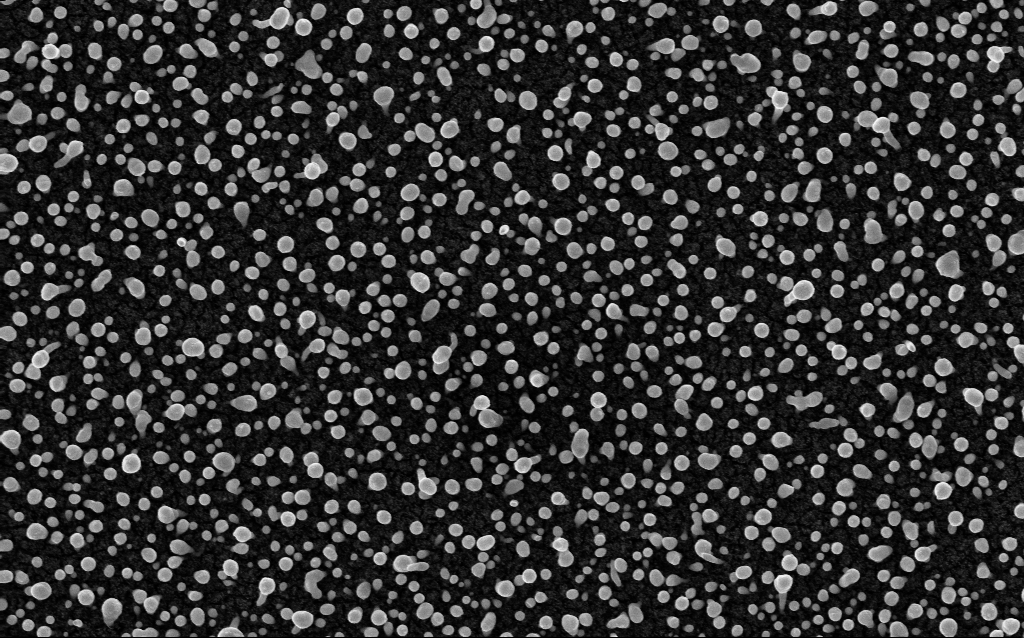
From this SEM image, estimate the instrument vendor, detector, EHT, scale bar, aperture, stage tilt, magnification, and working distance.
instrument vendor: Zeiss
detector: InLens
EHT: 5 kV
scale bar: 1000 nm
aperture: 30 µm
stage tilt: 0°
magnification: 50 K X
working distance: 2.1 mm